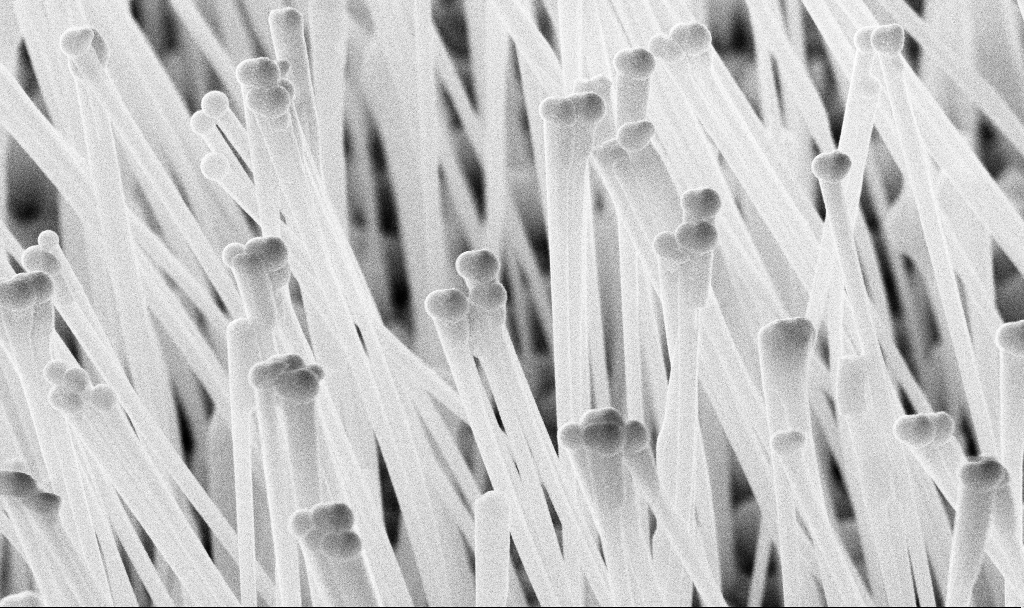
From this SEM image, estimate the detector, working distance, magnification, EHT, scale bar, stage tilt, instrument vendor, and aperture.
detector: InLens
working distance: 7.1 mm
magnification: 57.4 K X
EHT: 10 kV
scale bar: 1000 nm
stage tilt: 45°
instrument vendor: Zeiss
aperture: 30 µm